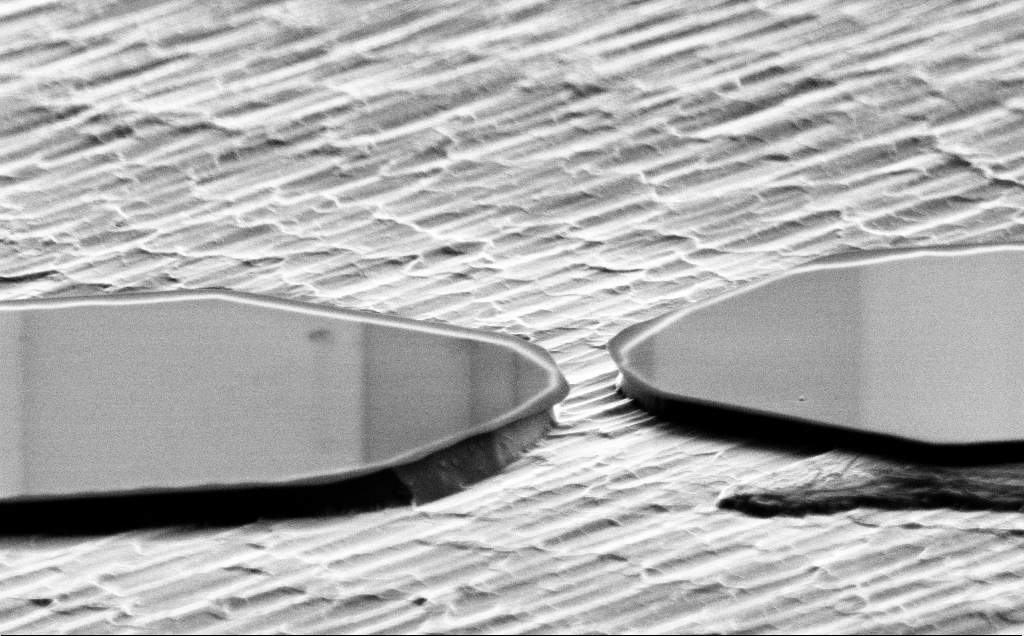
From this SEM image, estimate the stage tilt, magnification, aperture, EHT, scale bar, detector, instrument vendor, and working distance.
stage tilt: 70°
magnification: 11.32 K X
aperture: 30 µm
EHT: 5 kV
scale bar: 2000 nm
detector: SE2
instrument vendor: Zeiss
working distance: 10 mm